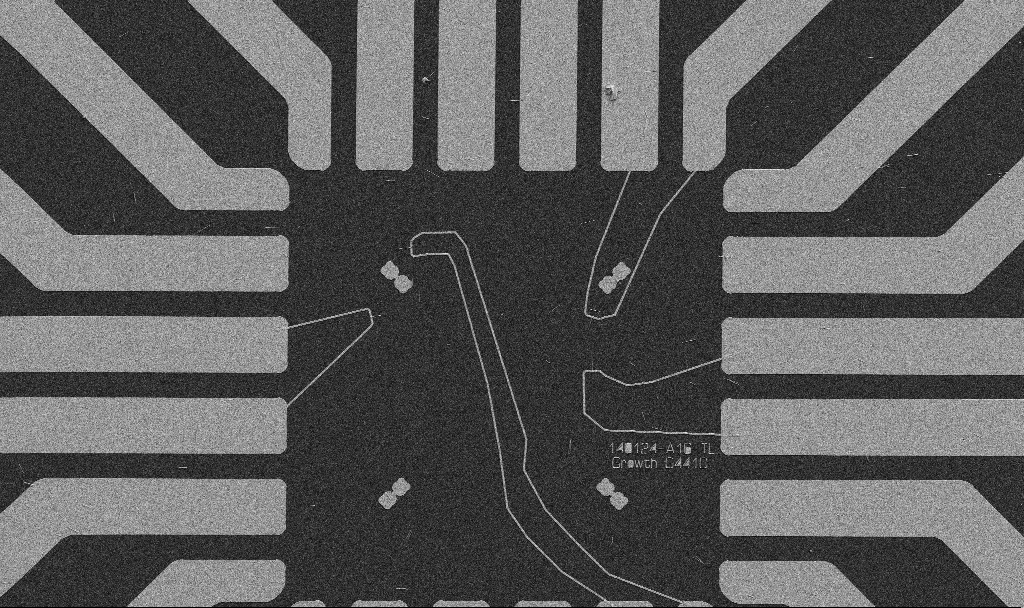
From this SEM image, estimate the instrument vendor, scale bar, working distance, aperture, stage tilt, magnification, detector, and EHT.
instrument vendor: Zeiss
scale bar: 20000 nm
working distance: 10.7 mm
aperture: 30 µm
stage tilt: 0°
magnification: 1 K X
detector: SE2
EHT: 5 kV